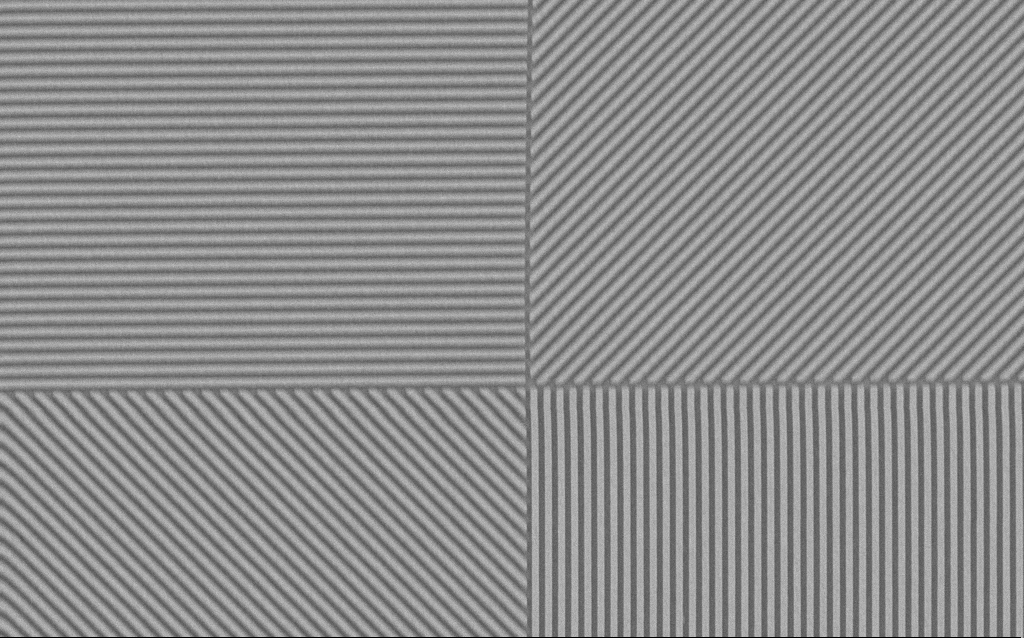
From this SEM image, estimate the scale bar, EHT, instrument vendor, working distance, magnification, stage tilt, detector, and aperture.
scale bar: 2000 nm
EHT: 3 kV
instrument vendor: Zeiss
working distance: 8.7 mm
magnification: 11.98 K X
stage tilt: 0°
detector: SE2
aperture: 30 µm